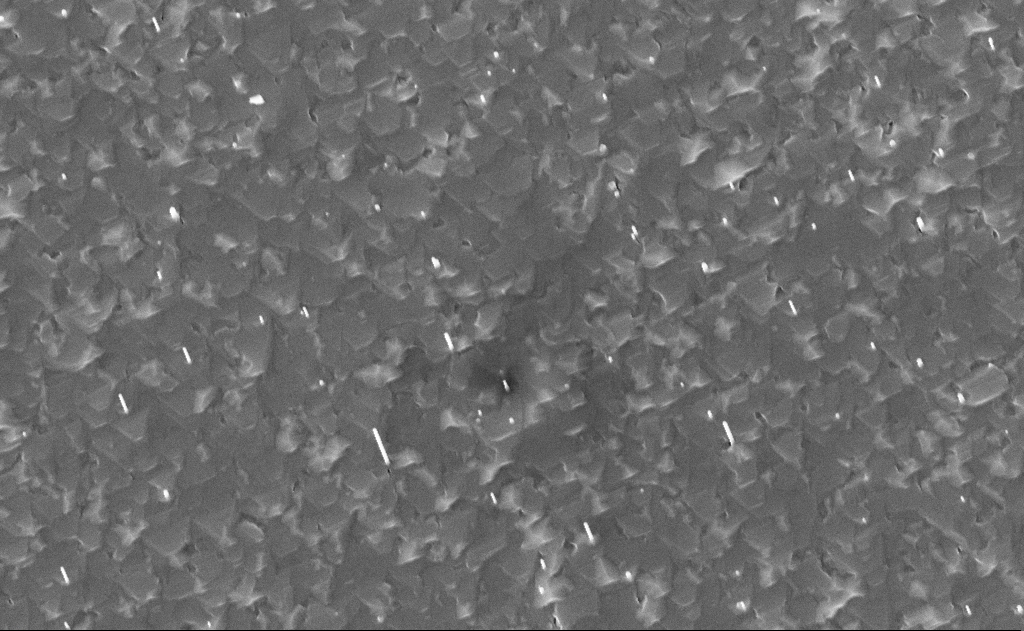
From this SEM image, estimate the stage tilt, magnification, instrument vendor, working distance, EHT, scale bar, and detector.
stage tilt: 0°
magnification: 30 K X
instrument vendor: Zeiss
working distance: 14 mm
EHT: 10 kV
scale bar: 1000 nm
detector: InLens